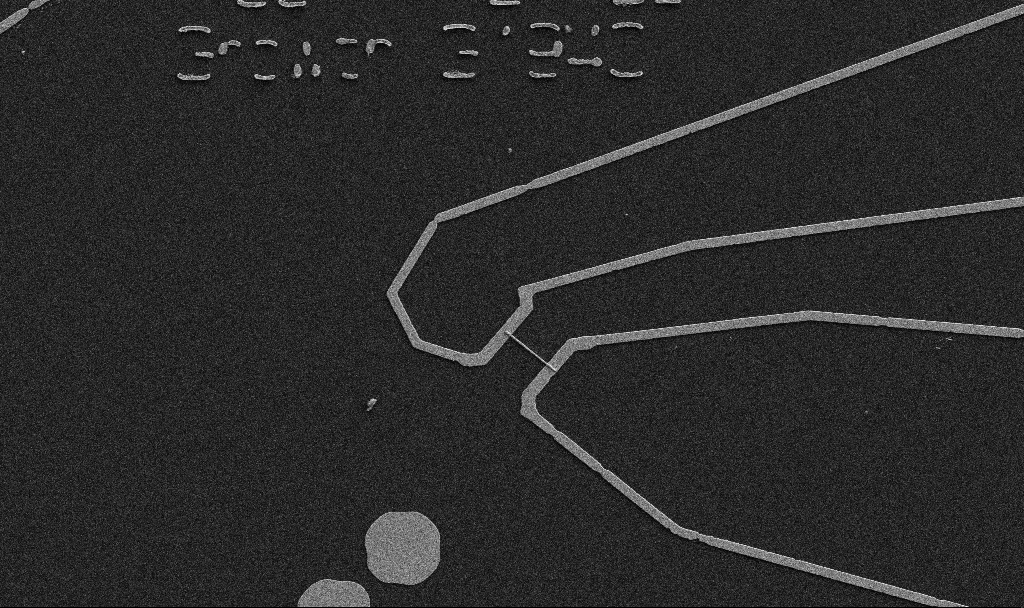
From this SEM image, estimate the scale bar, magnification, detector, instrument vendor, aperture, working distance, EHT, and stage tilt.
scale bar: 10000 nm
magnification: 5 K X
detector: SE2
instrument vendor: Zeiss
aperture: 30 µm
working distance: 10.7 mm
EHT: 5 kV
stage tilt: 0°